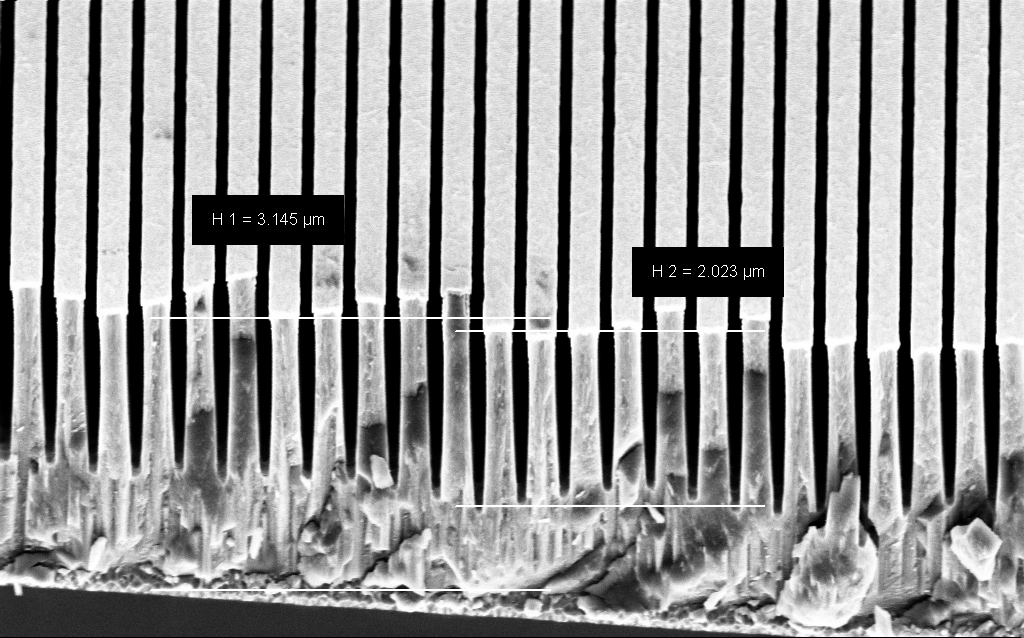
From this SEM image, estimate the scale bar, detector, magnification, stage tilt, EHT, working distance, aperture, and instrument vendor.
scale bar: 1000 nm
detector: InLens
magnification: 31.76 K X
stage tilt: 45°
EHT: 3 kV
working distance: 6 mm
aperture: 30 µm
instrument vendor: Zeiss